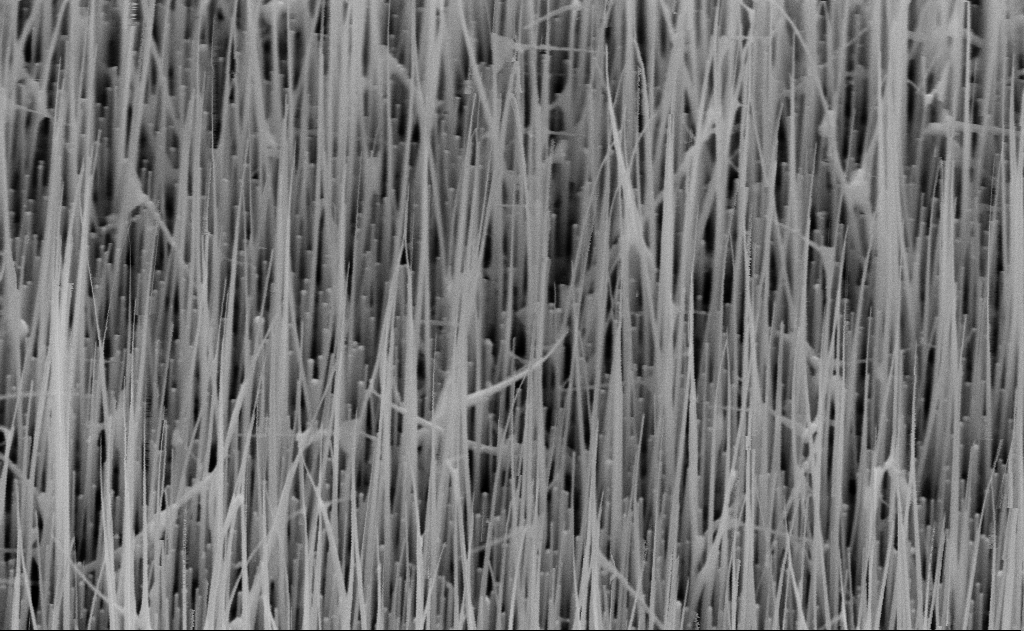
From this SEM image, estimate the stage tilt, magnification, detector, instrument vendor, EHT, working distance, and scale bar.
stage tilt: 45°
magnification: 40 K X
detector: SE2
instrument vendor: Zeiss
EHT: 10 kV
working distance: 16 mm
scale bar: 1000 nm